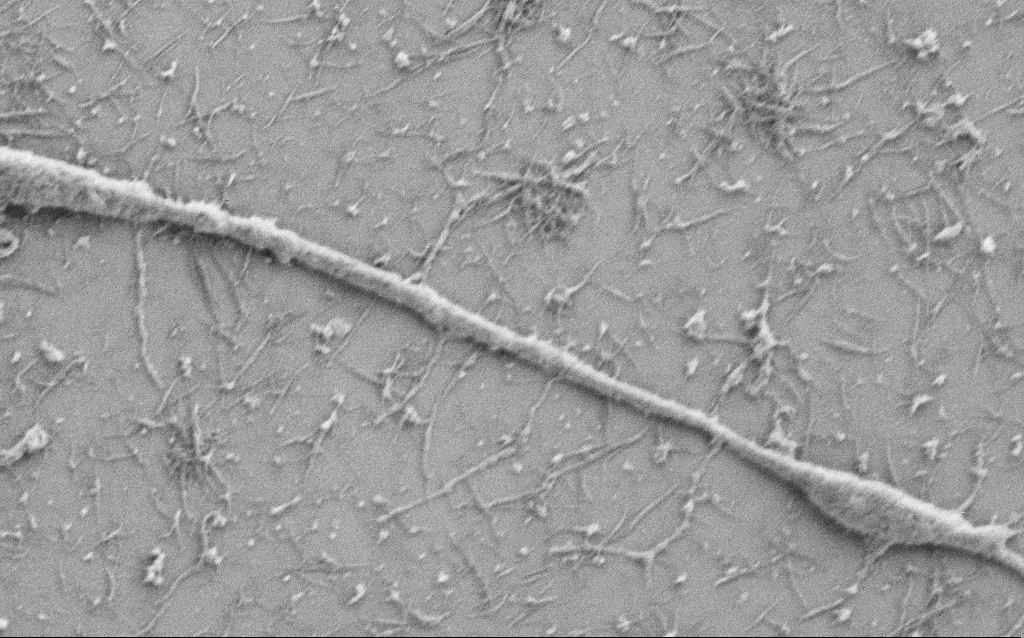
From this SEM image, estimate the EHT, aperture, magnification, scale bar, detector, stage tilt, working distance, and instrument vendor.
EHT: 1 kV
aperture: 30 µm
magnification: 25 K X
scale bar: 1000 nm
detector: SE2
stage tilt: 0°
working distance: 6.8 mm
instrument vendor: Zeiss